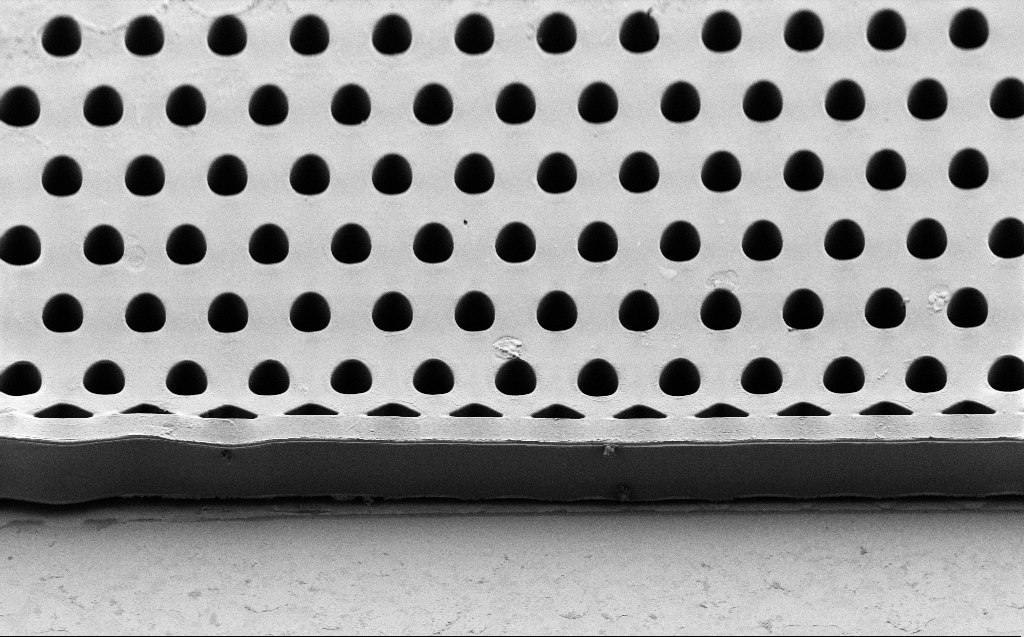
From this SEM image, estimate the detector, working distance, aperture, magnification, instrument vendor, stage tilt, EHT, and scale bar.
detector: SE2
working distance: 6 mm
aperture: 30 µm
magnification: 1.25 K X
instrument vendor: Zeiss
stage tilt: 45°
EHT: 2 kV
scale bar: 20000 nm